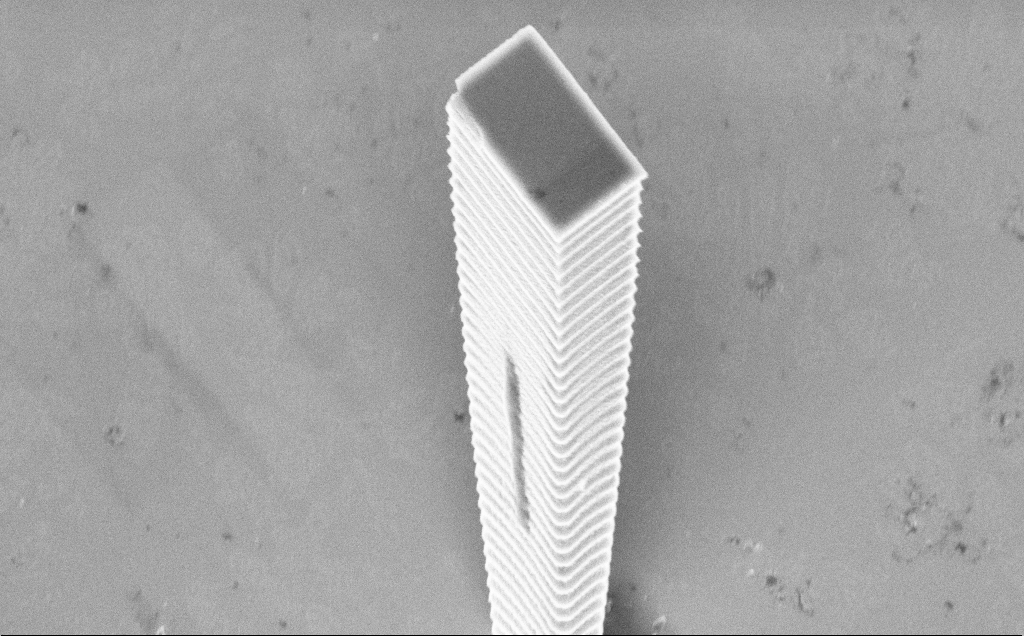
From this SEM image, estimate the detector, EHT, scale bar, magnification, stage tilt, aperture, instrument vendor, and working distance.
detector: InLens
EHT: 5 kV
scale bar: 2000 nm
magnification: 14.31 K X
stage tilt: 45°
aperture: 30 µm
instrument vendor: Zeiss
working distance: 14 mm